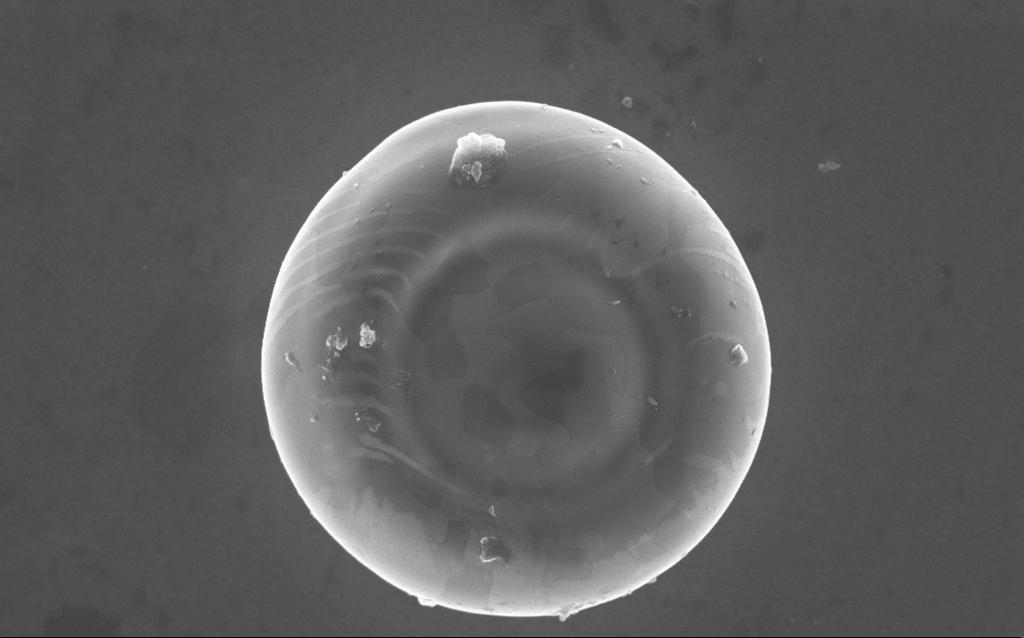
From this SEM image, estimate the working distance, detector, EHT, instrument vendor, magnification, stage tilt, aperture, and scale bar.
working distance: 2 mm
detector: InLens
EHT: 5 kV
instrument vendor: Zeiss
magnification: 50 K X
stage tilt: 0°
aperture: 30 µm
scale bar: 1000 nm